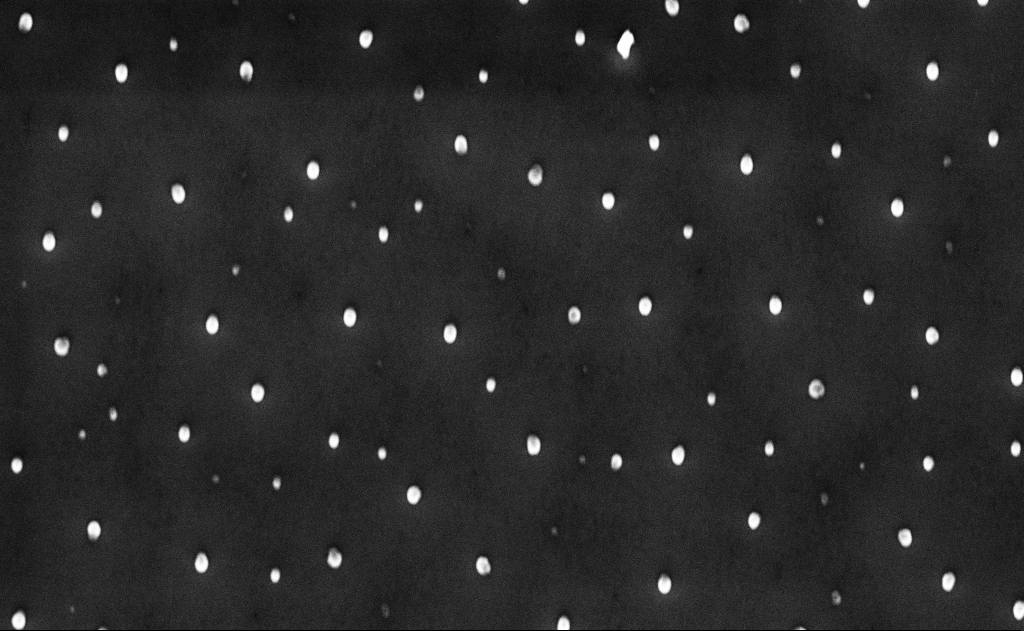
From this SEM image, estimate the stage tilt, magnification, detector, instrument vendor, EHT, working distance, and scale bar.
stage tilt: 0°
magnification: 10 K X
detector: InLens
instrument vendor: Zeiss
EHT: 10 kV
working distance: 10 mm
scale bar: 2000 nm